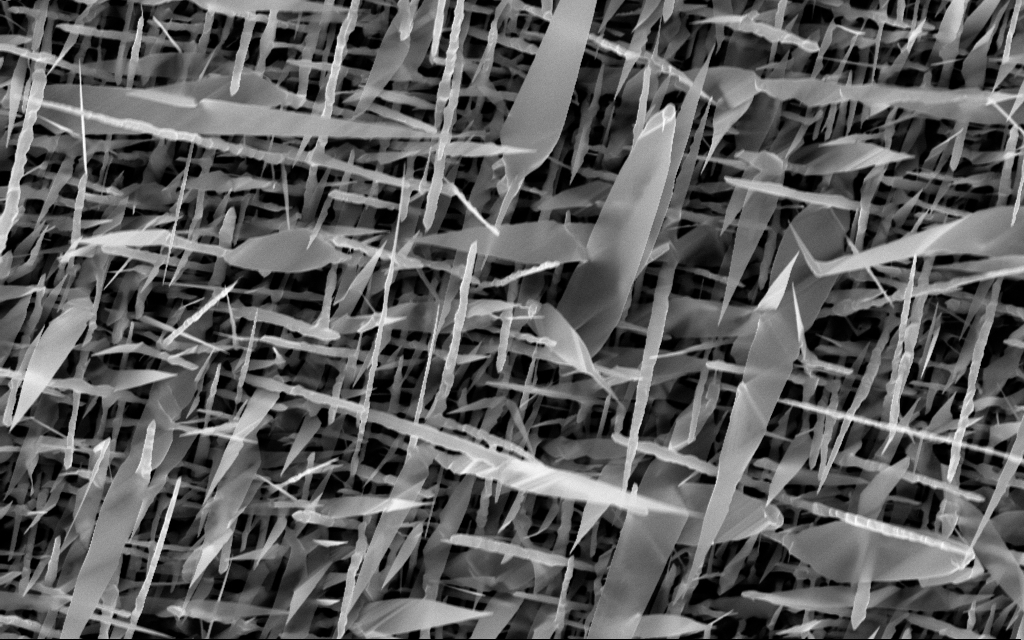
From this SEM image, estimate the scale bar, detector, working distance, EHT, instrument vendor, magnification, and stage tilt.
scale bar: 2000 nm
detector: InLens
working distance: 7 mm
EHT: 10 kV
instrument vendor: Zeiss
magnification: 20 K X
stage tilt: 0°